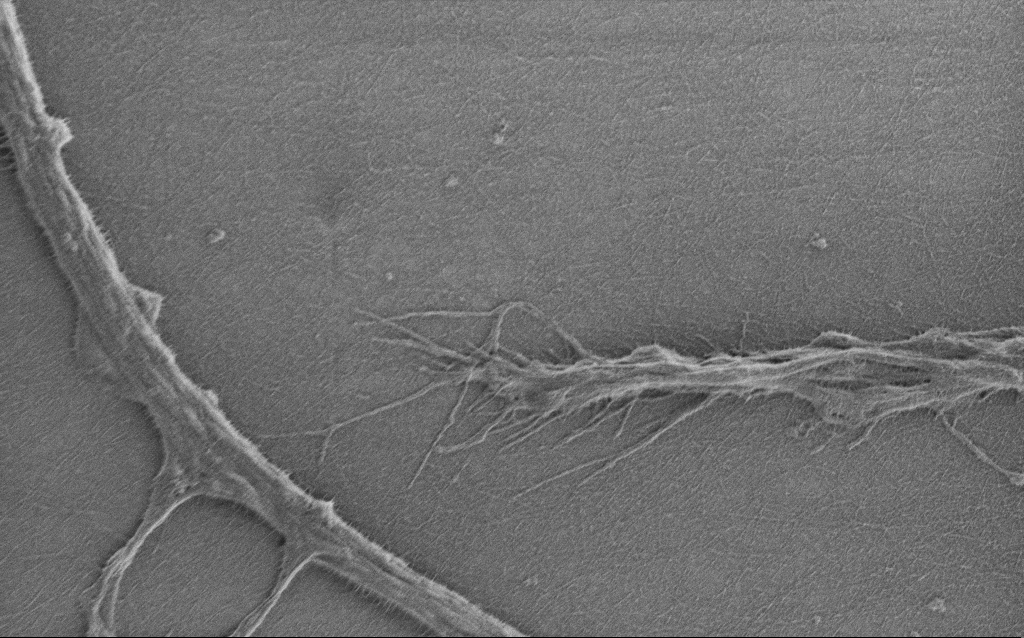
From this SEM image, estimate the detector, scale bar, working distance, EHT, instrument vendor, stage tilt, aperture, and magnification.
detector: SE2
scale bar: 2000 nm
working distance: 6 mm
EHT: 0.9 kV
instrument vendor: Zeiss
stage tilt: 0°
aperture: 30 µm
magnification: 10 K X